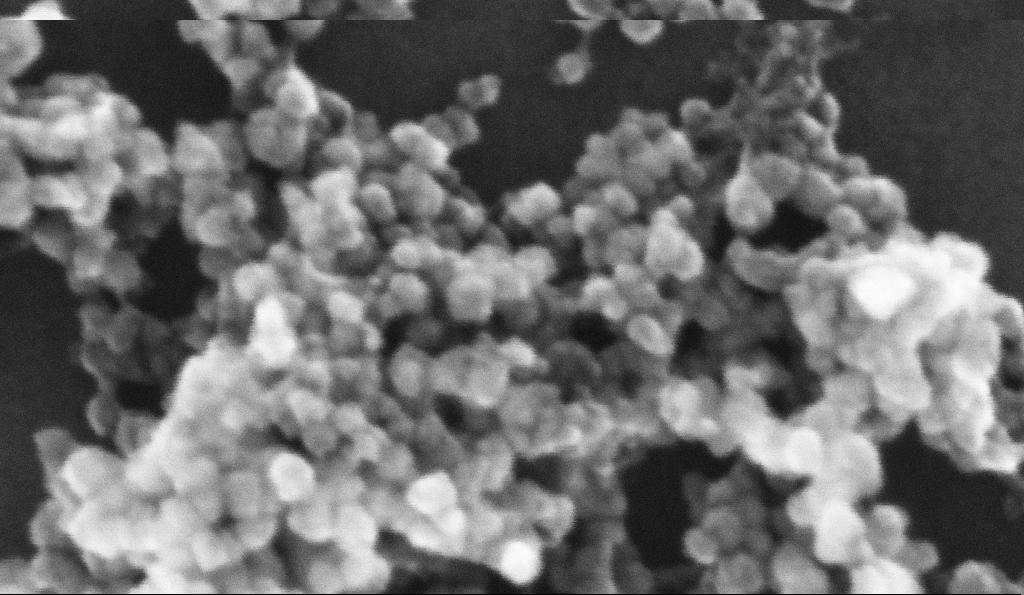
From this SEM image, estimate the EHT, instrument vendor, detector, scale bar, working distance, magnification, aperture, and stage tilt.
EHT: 10 kV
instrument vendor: Zeiss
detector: InLens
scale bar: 20 nm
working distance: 5.2 mm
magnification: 765.74 K X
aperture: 30 µm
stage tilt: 0°